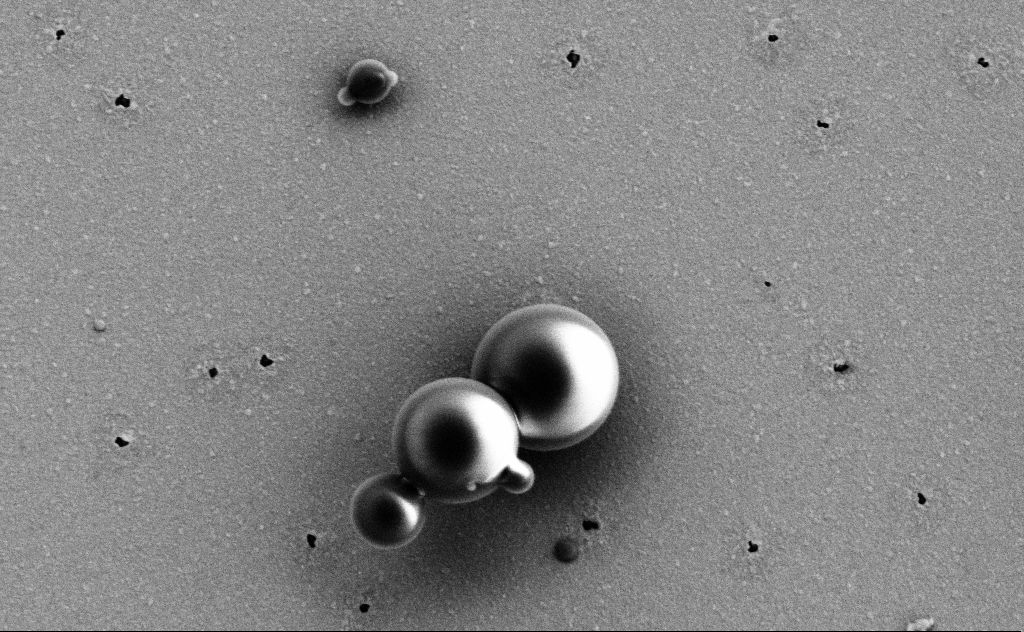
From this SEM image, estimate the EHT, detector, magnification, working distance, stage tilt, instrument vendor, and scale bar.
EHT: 3 kV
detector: SE2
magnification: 24.86 K X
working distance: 10 mm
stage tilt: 0°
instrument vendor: Zeiss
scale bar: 2000 nm